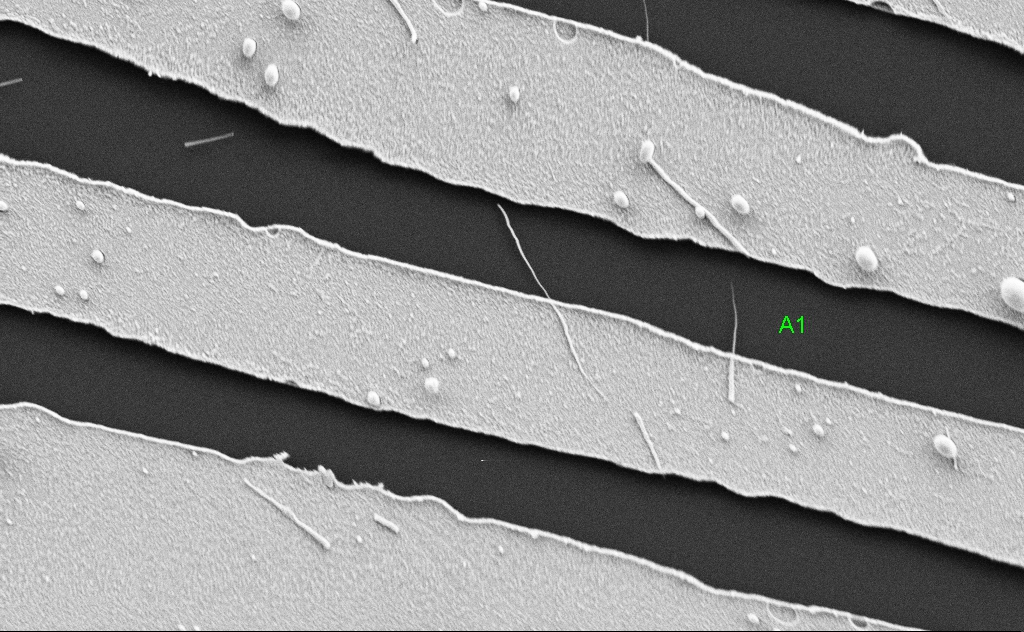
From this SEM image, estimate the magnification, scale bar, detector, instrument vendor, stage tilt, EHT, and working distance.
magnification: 20.03 K X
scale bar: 1000 nm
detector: SE2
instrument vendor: Zeiss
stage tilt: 0°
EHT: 5 kV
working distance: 5 mm